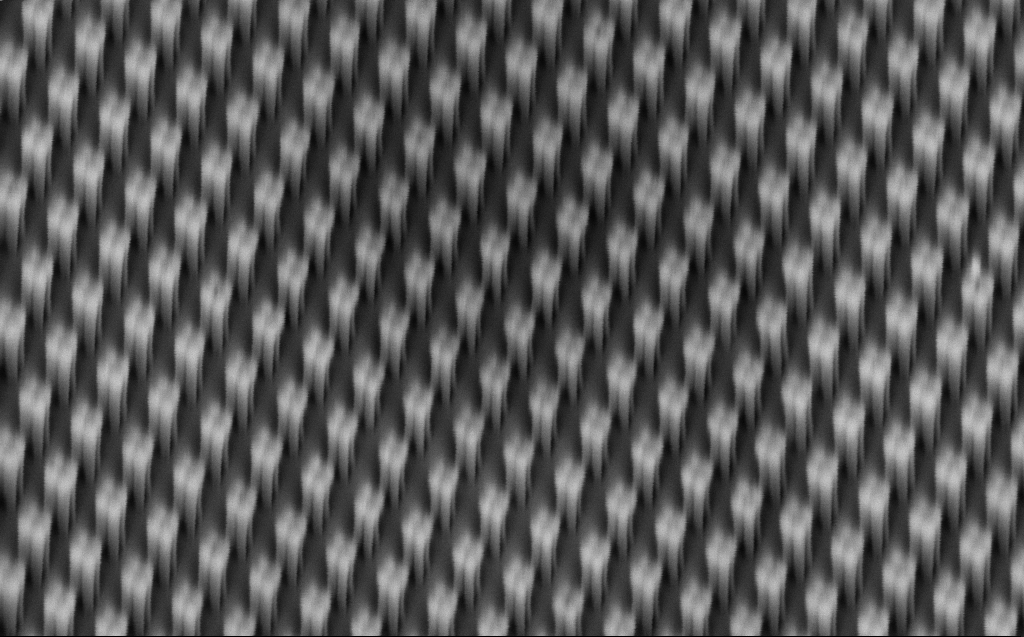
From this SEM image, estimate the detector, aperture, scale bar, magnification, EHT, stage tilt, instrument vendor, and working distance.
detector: InLens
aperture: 30 µm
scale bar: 1000 nm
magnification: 42.28 K X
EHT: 1 kV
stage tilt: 42.8°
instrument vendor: Zeiss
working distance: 6 mm